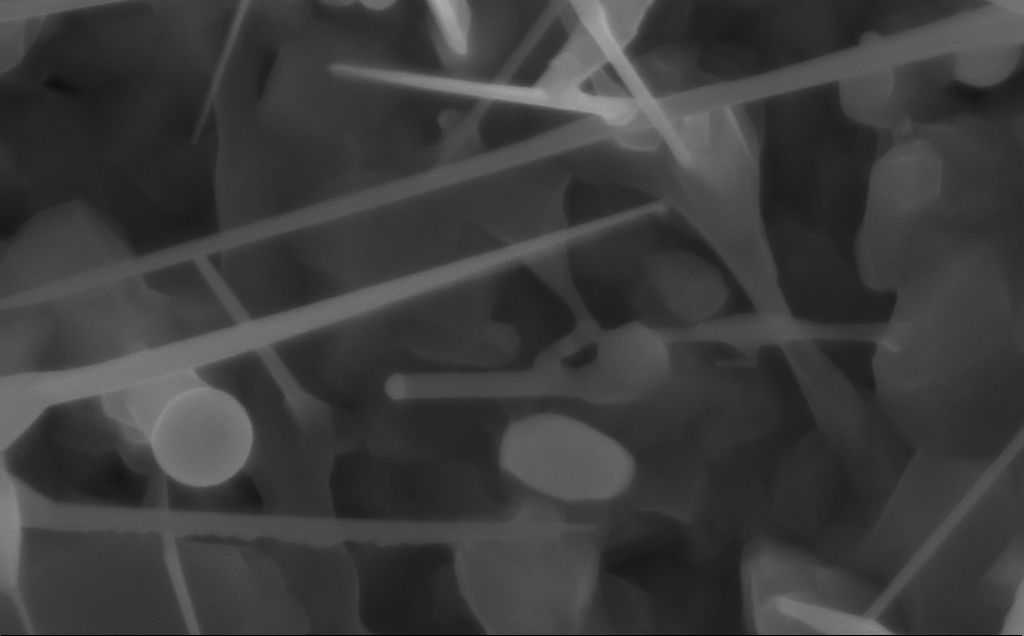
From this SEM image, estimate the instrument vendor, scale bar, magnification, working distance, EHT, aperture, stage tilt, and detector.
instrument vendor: Zeiss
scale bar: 100 nm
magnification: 150 K X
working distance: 3 mm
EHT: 10 kV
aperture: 30 µm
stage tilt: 0°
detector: InLens